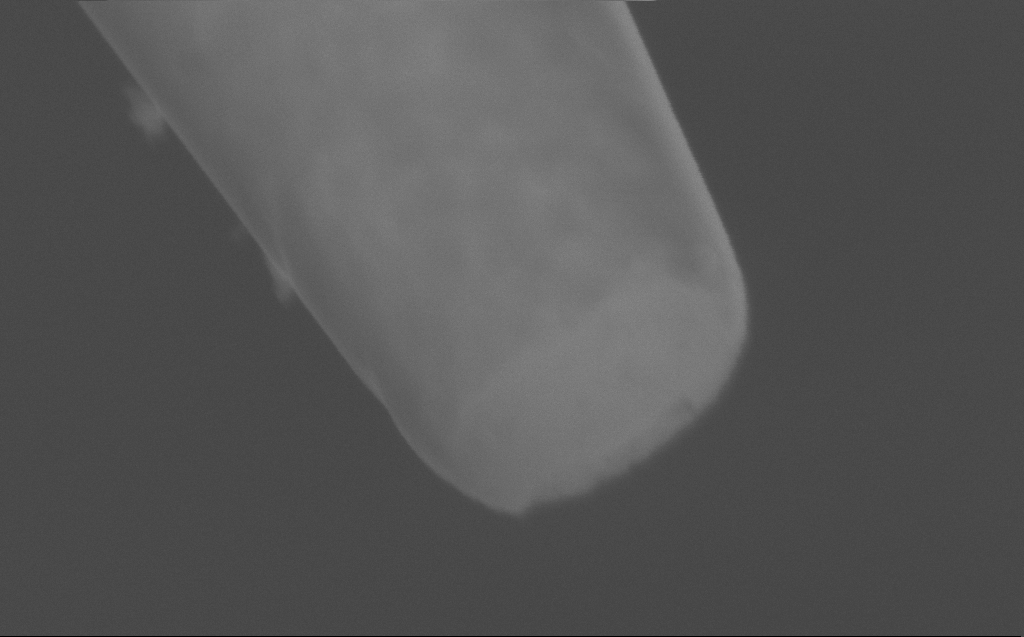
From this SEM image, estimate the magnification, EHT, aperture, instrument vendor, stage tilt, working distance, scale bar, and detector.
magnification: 171.97 K X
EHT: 5 kV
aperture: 30 µm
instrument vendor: Zeiss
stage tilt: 45°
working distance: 4 mm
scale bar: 200 nm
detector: SE2